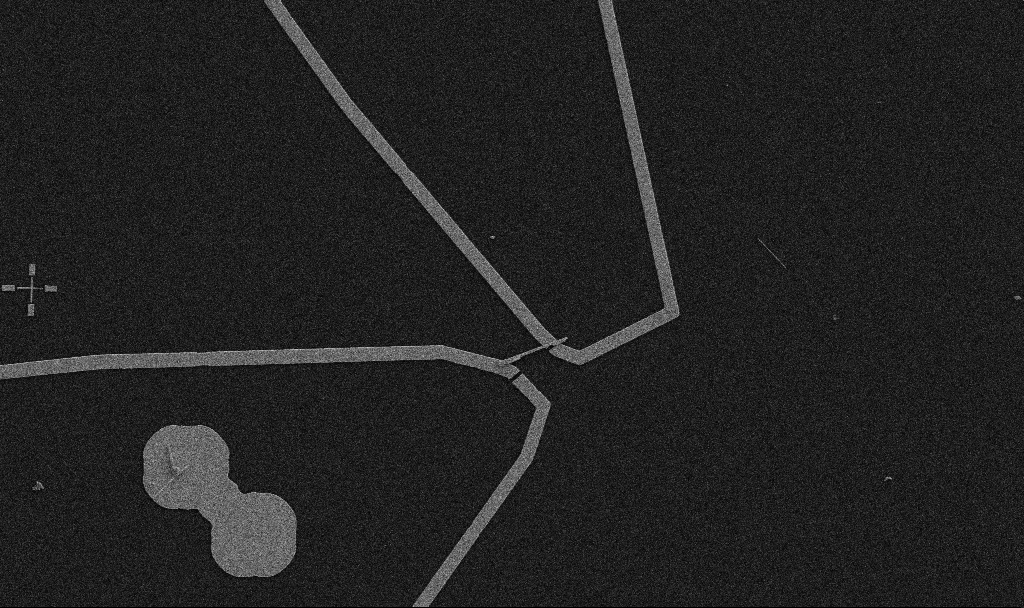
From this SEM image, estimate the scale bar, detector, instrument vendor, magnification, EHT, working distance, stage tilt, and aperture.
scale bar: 10000 nm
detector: SE2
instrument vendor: Zeiss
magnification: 5 K X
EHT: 5 kV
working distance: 10.7 mm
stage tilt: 0°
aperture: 30 µm